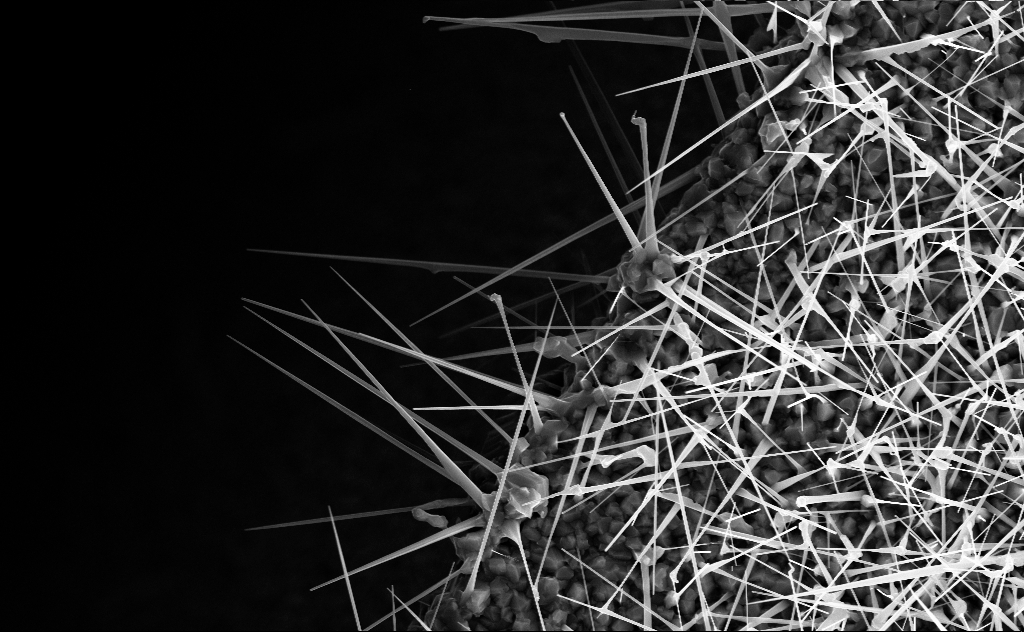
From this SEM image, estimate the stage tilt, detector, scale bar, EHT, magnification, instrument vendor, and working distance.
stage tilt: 0°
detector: InLens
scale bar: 2000 nm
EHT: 10 kV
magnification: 16.38 K X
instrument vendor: Zeiss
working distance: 6 mm